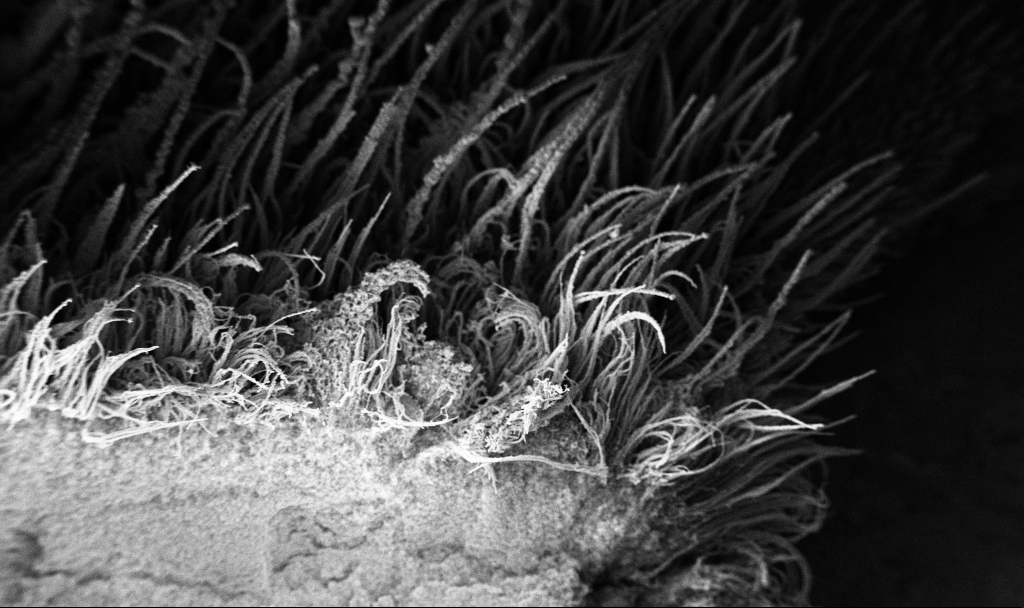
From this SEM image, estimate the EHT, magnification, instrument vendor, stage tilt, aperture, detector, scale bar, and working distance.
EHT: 3 kV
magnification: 0.15 K X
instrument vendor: Zeiss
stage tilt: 37.7°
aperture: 30 µm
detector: InLens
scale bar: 100000 nm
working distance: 7.5 mm